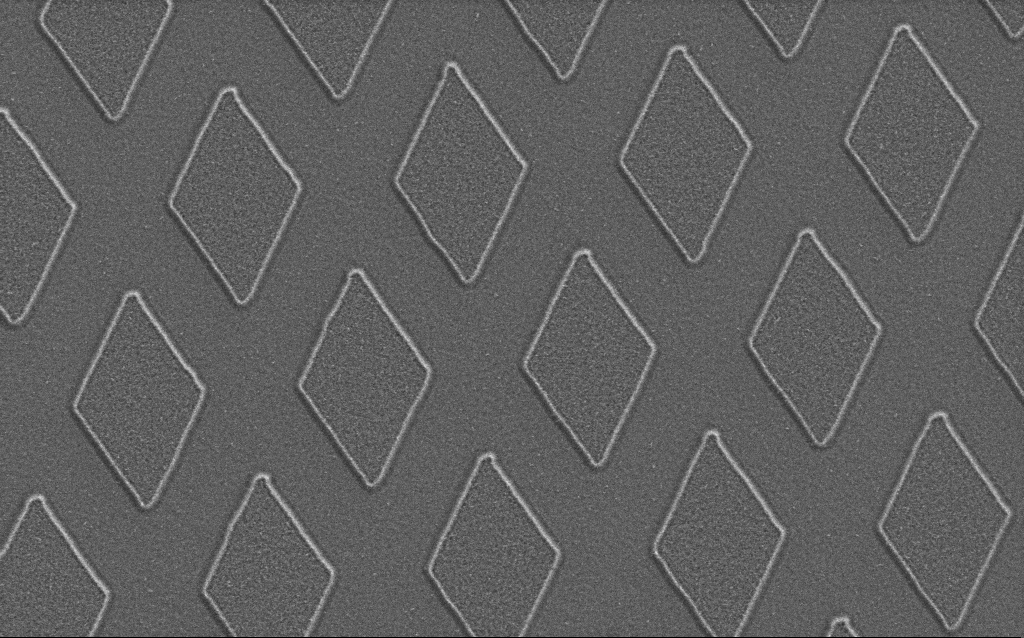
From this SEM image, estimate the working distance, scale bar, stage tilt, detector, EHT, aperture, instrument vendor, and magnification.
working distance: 5 mm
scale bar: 2000 nm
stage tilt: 0°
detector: SE2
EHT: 1.5 kV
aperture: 30 µm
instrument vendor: Zeiss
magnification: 28.56 K X